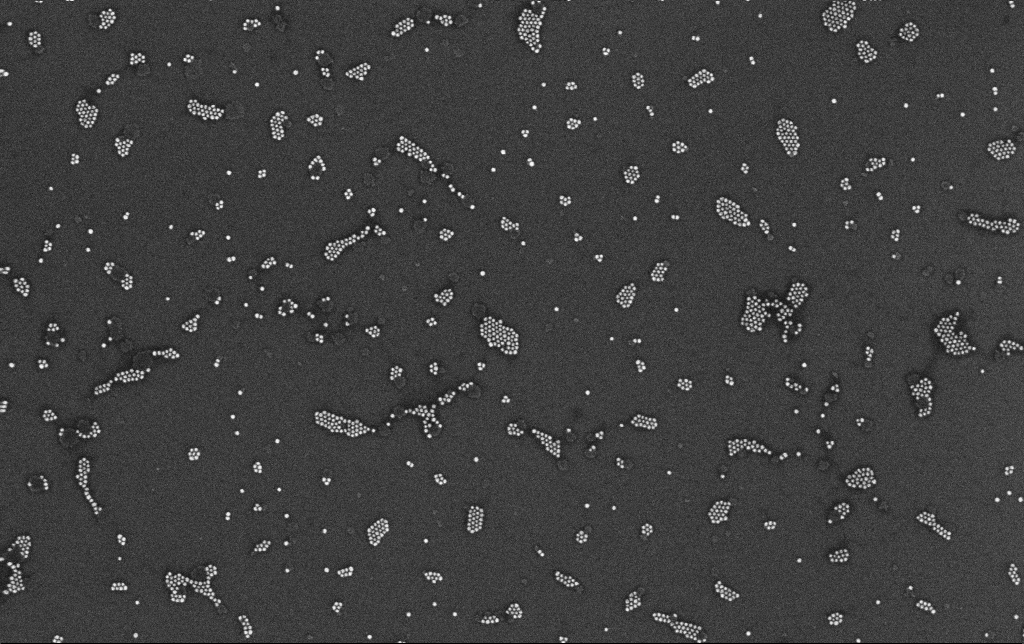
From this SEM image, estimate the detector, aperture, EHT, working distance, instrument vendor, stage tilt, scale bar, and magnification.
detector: InLens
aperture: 30 µm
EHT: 10 kV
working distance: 3.4 mm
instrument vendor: Zeiss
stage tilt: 0°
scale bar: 200 nm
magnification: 100 K X